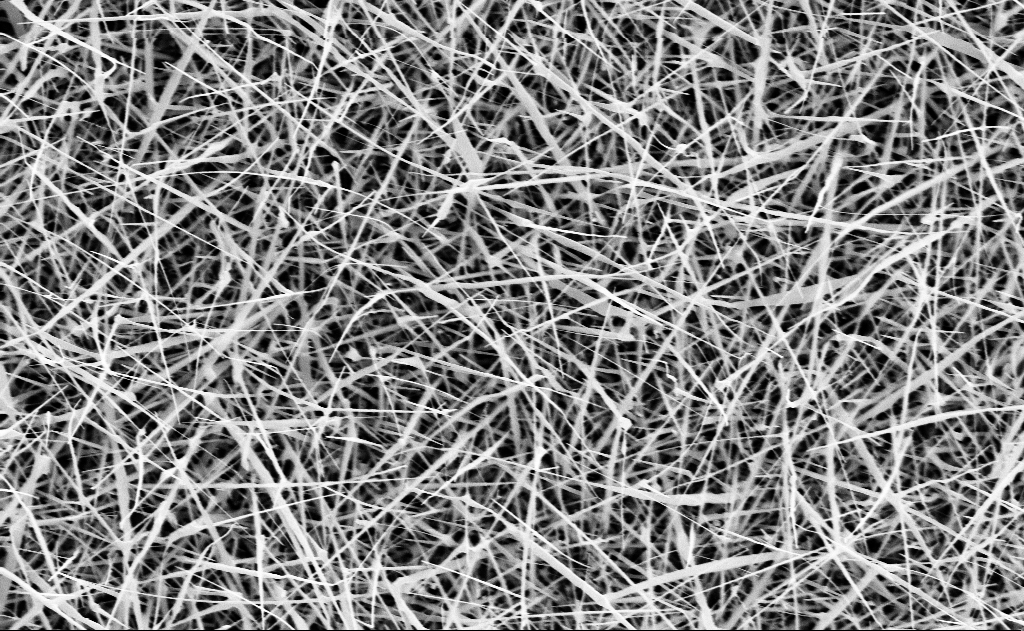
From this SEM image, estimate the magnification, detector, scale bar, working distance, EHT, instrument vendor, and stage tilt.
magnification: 20 K X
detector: InLens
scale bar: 1000 nm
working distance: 15 mm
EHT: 10 kV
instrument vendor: Zeiss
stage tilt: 0°